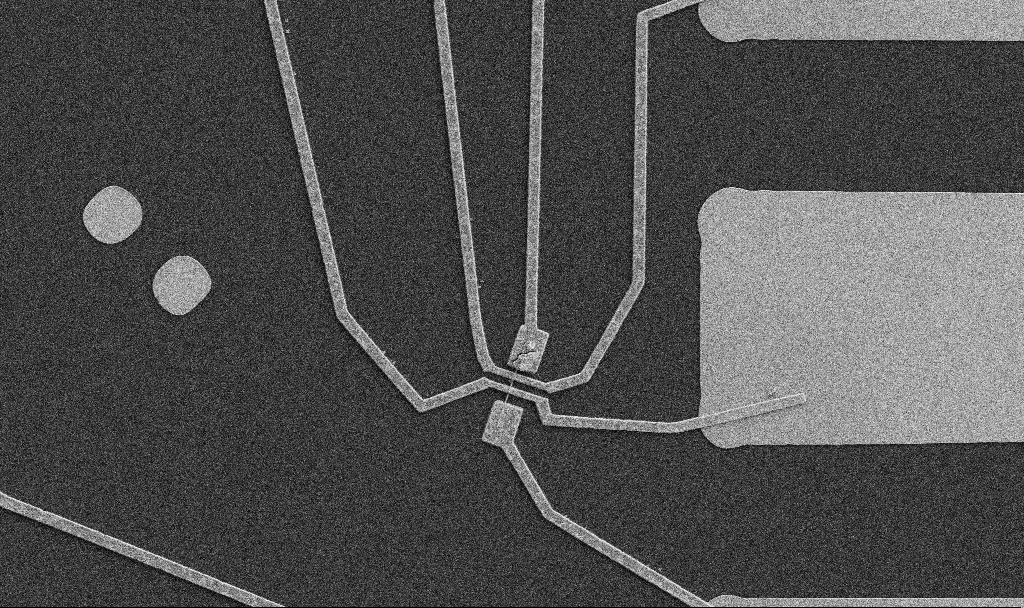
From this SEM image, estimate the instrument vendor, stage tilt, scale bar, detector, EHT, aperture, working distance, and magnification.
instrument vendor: Zeiss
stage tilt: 0°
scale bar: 10000 nm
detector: SE2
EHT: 5 kV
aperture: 30 µm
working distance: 8.7 mm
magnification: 5 K X